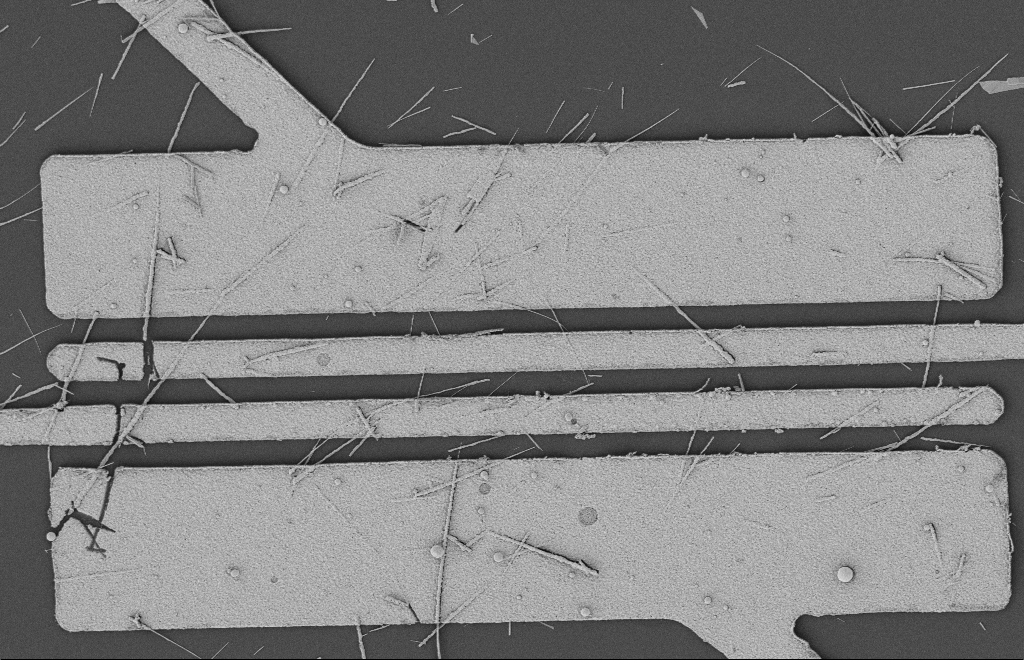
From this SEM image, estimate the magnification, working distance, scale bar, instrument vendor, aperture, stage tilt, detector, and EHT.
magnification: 5.73 K X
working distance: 12 mm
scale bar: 2000 nm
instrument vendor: Zeiss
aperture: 20 µm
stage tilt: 0°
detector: SE2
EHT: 2 kV